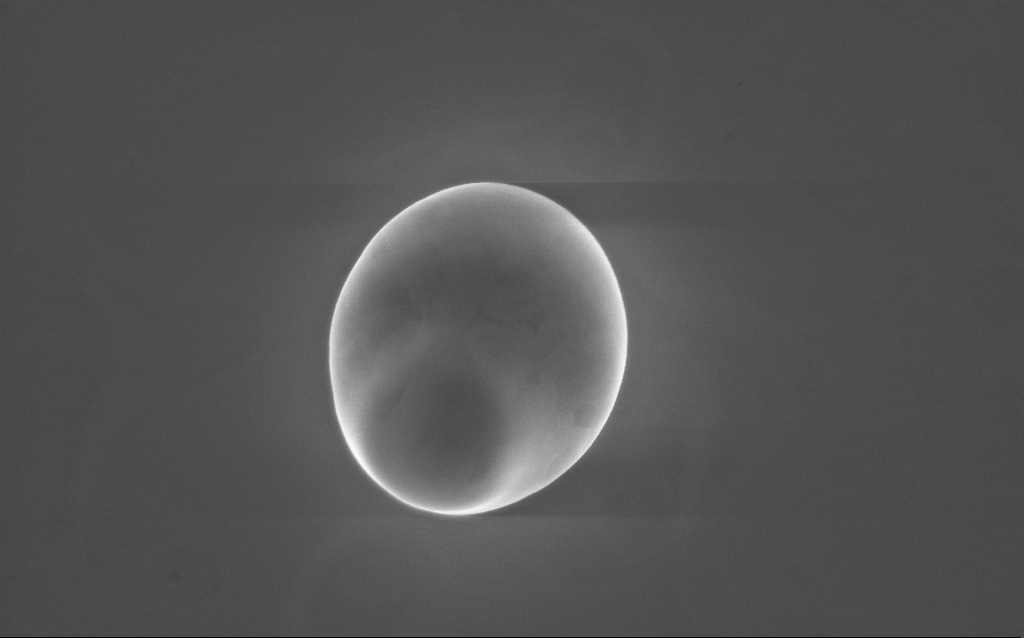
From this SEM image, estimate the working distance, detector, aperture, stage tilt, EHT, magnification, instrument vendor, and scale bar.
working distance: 2 mm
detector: InLens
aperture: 30 µm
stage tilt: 0°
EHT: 10 kV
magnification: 42.43 K X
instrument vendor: Zeiss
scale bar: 1000 nm